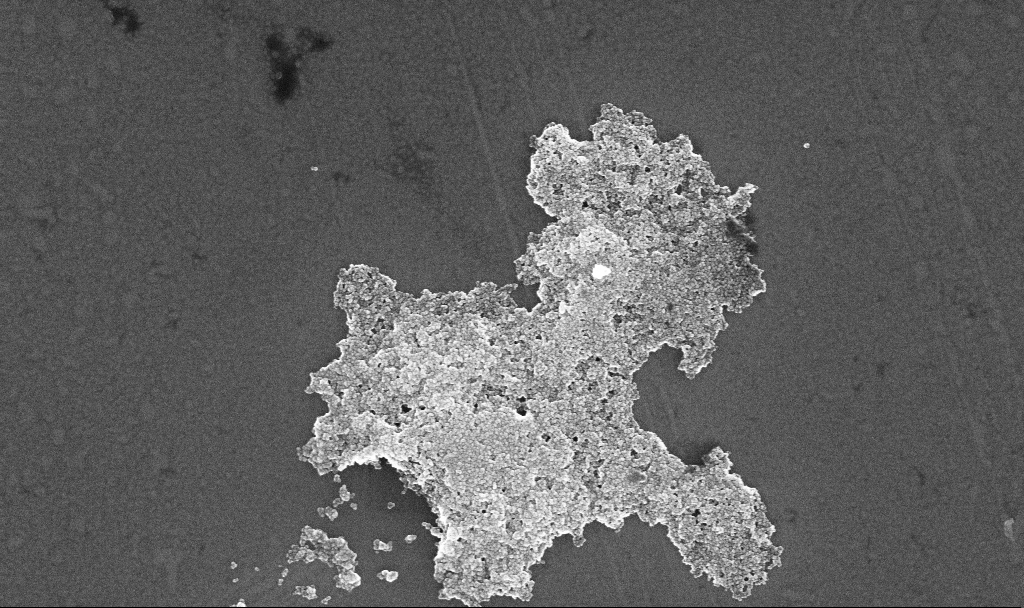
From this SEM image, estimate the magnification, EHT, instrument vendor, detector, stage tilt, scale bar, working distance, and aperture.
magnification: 47.46 K X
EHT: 10 kV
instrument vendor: Zeiss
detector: InLens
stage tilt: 0°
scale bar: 1000 nm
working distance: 5.2 mm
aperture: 30 µm